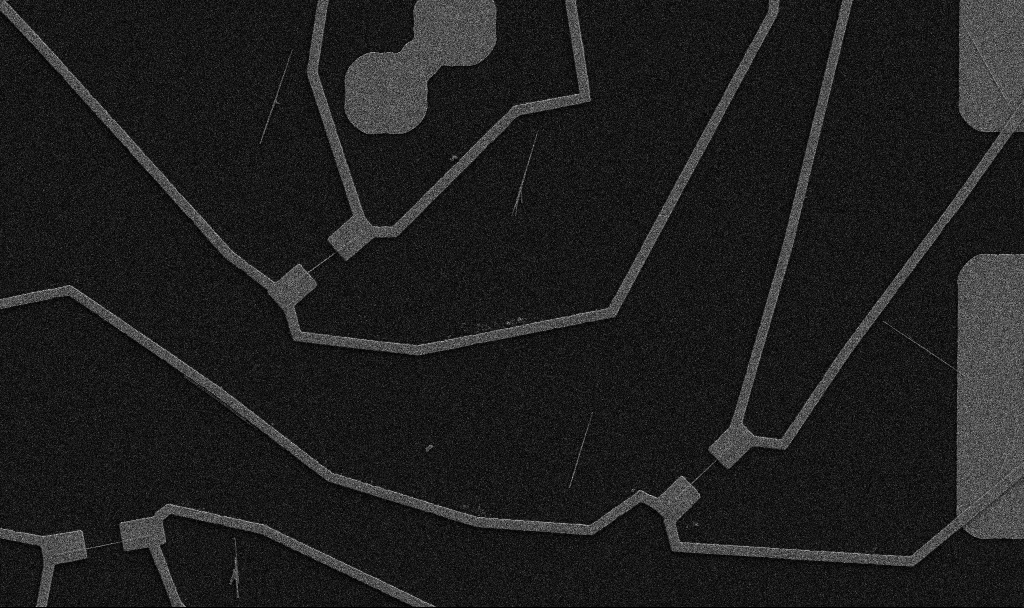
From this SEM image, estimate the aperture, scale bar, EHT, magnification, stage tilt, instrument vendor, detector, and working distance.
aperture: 30 µm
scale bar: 10000 nm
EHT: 5 kV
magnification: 5 K X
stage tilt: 0°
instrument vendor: Zeiss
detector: SE2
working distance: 10.7 mm